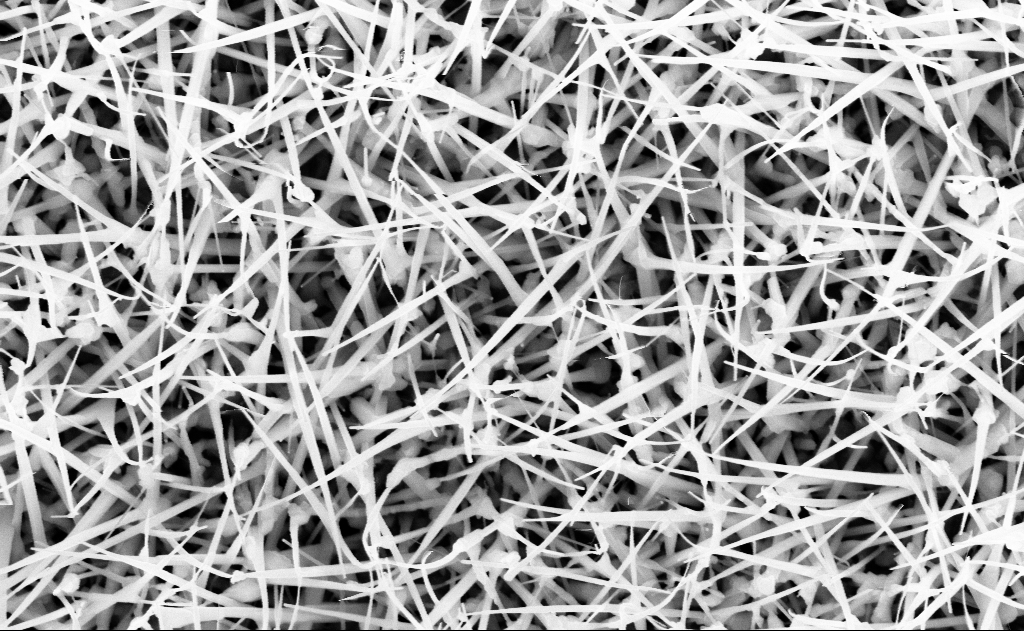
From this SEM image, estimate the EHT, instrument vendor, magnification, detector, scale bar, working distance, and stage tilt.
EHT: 10 kV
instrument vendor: Zeiss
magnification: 40 K X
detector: InLens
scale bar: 1000 nm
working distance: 13 mm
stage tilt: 0°